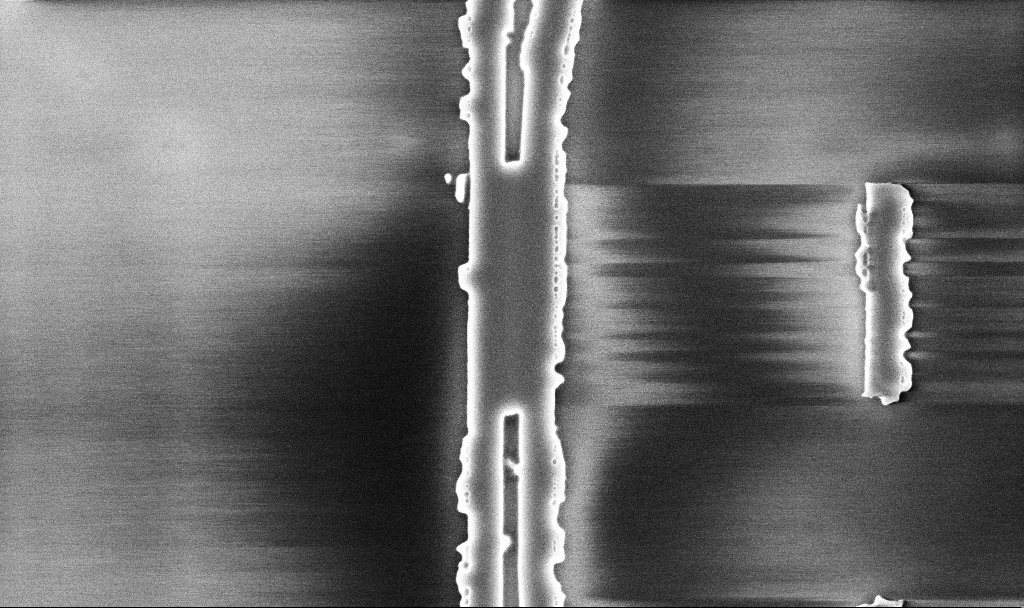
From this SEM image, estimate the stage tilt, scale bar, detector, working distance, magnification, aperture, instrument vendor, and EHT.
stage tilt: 0°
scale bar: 1000 nm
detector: InLens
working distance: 10.1 mm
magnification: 26.16 K X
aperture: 30 µm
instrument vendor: Zeiss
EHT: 5 kV